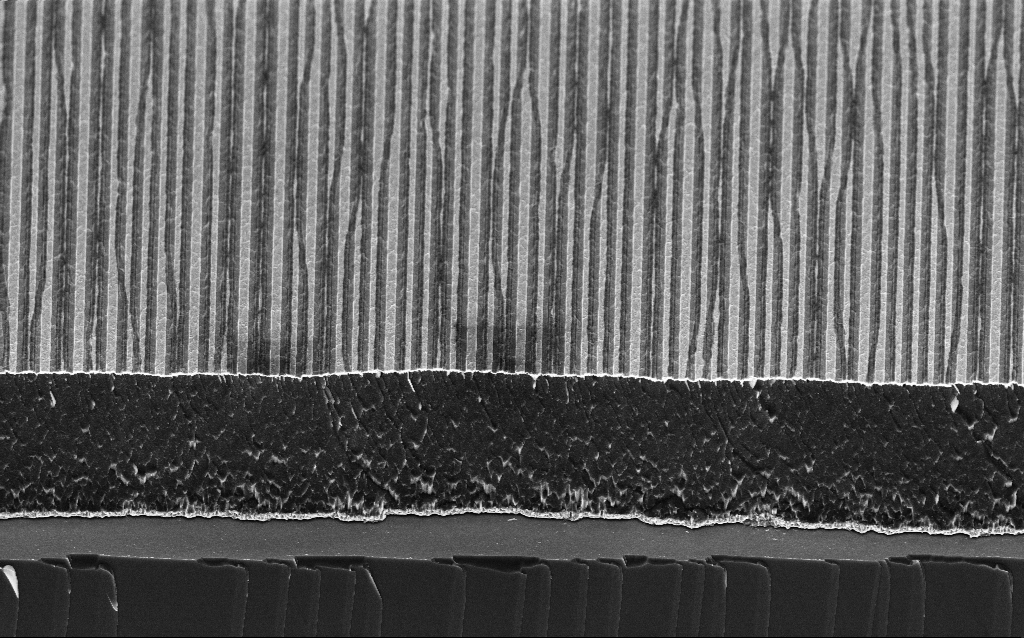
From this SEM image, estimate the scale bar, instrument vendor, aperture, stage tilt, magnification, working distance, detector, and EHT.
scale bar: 1000 nm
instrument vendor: Zeiss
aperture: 30 µm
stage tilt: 45°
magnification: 12.16 K X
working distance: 6 mm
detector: InLens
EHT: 3 kV